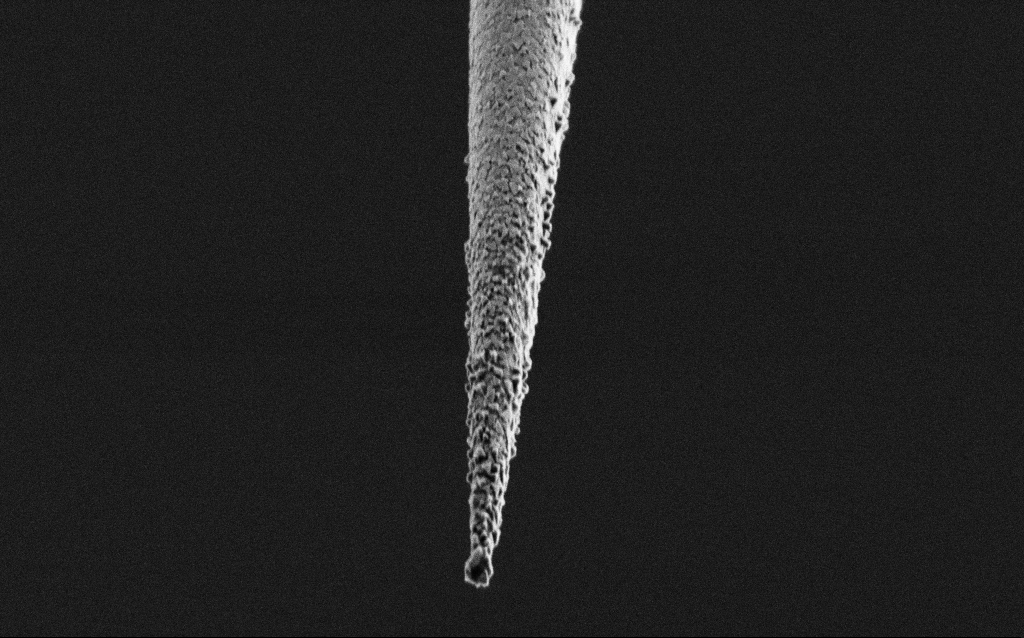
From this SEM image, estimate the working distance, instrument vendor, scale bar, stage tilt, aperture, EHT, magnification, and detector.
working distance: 6.3 mm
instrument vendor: Zeiss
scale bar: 2000 nm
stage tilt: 44.9°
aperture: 30 µm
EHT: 2 kV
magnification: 25 K X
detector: SE2